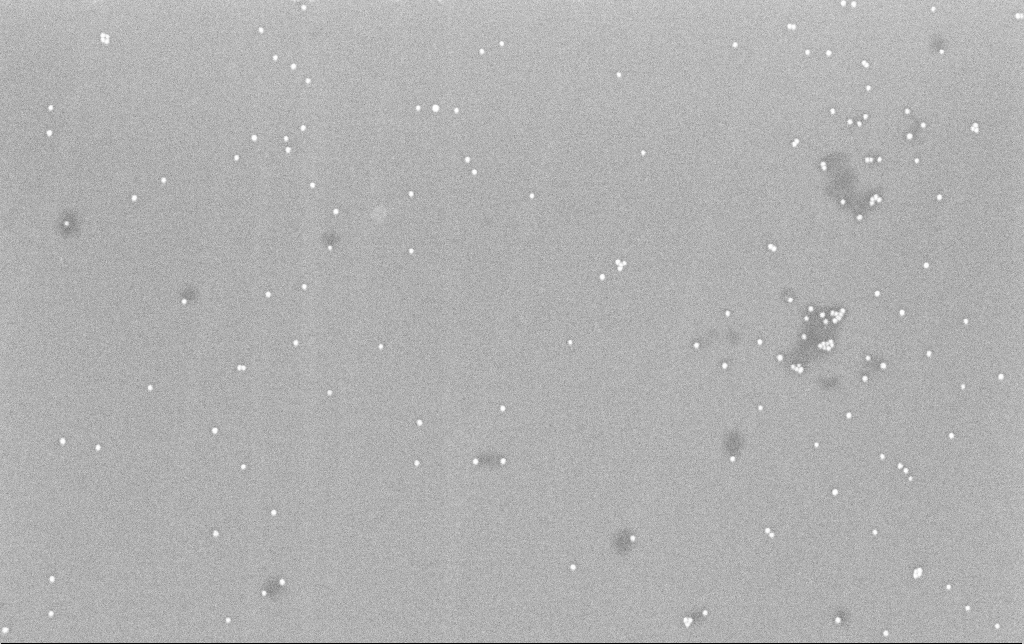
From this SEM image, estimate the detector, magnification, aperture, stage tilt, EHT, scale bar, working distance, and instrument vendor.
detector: InLens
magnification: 100 K X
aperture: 30 µm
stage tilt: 0°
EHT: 8 kV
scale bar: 200 nm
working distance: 6.6 mm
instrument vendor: Zeiss